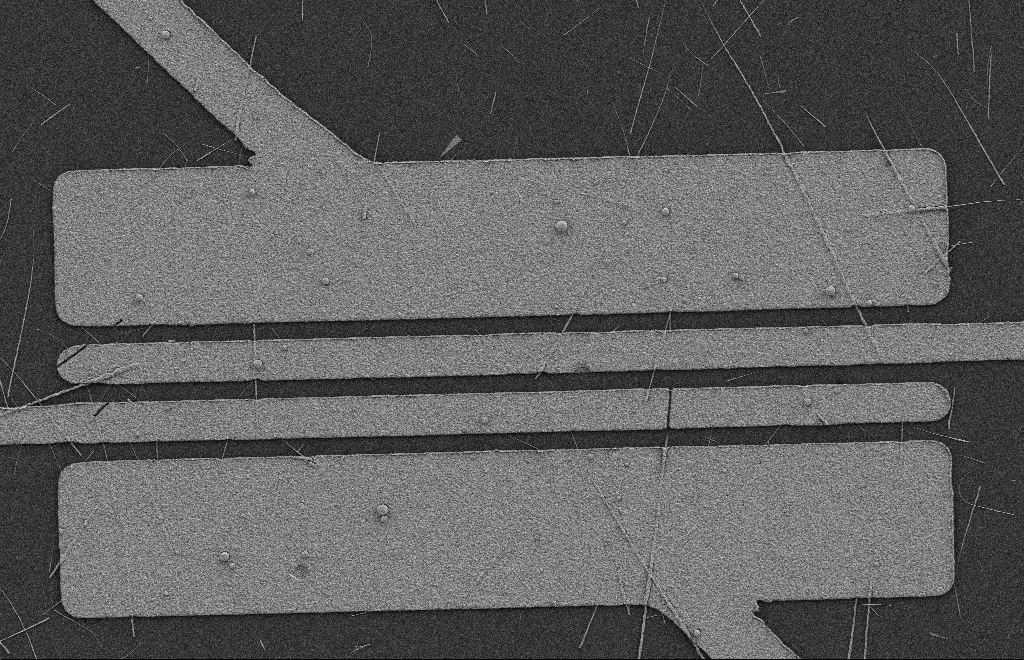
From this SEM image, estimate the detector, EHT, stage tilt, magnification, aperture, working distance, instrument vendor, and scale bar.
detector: SE2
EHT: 2 kV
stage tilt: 0°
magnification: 5.37 K X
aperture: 20 µm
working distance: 9 mm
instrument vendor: Zeiss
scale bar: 2000 nm